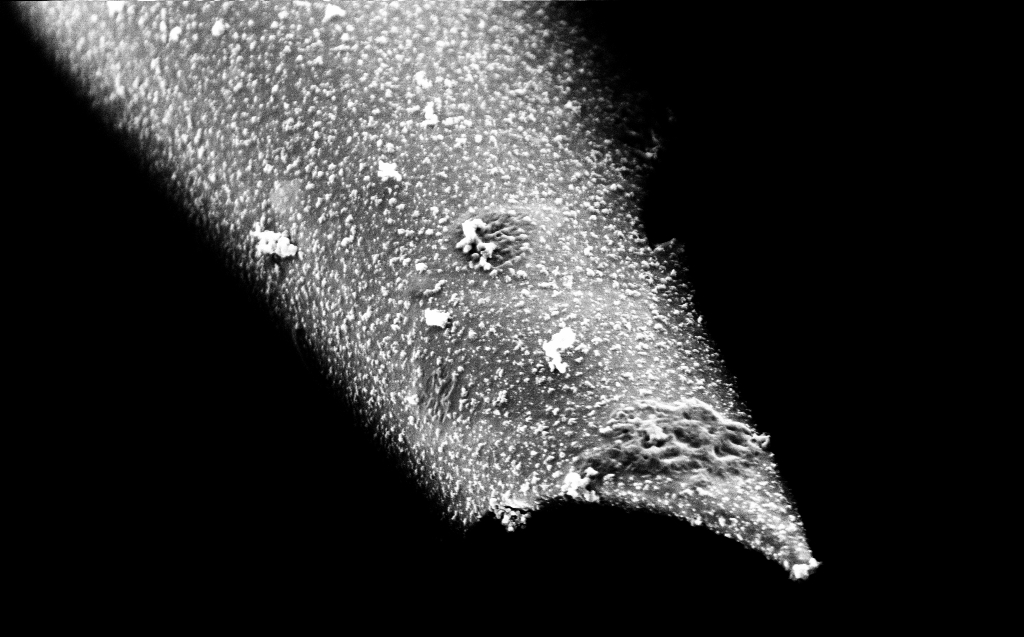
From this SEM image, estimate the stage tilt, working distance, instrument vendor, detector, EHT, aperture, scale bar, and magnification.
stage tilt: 45°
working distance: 4 mm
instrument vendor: Zeiss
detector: InLens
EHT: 2 kV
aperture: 30 µm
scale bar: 1000 nm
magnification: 50 K X